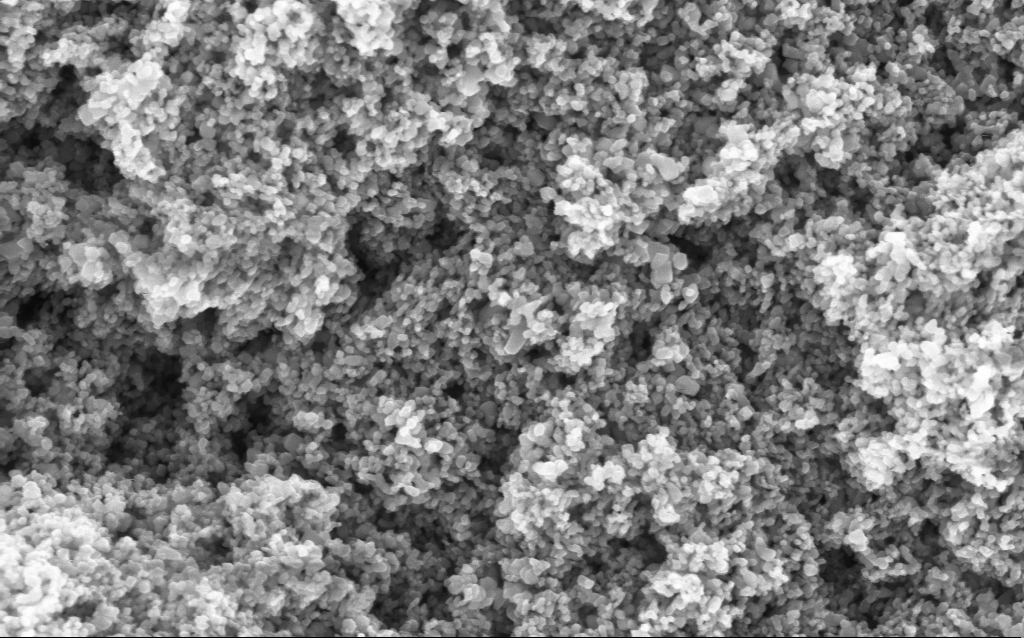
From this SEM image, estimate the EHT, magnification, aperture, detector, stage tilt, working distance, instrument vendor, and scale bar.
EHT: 5 kV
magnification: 114.64 K X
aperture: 30 µm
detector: InLens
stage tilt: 0°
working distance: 4.5 mm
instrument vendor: Zeiss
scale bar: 200 nm